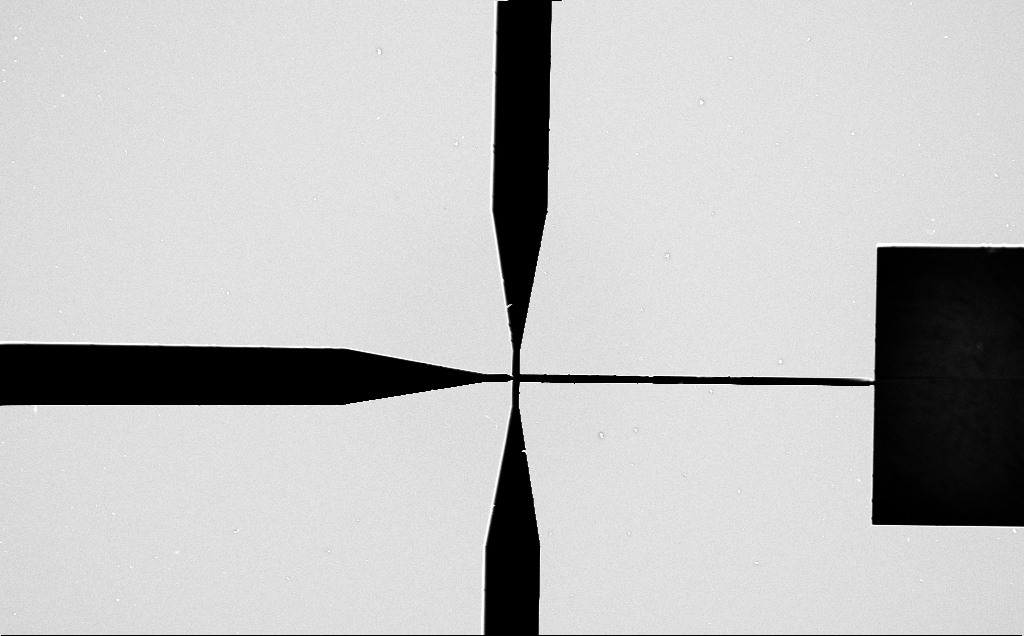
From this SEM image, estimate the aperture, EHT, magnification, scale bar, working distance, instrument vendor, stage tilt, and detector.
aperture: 30 µm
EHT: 5 kV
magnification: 0.262 K X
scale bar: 100000 nm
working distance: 7 mm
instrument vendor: Zeiss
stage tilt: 0°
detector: InLens